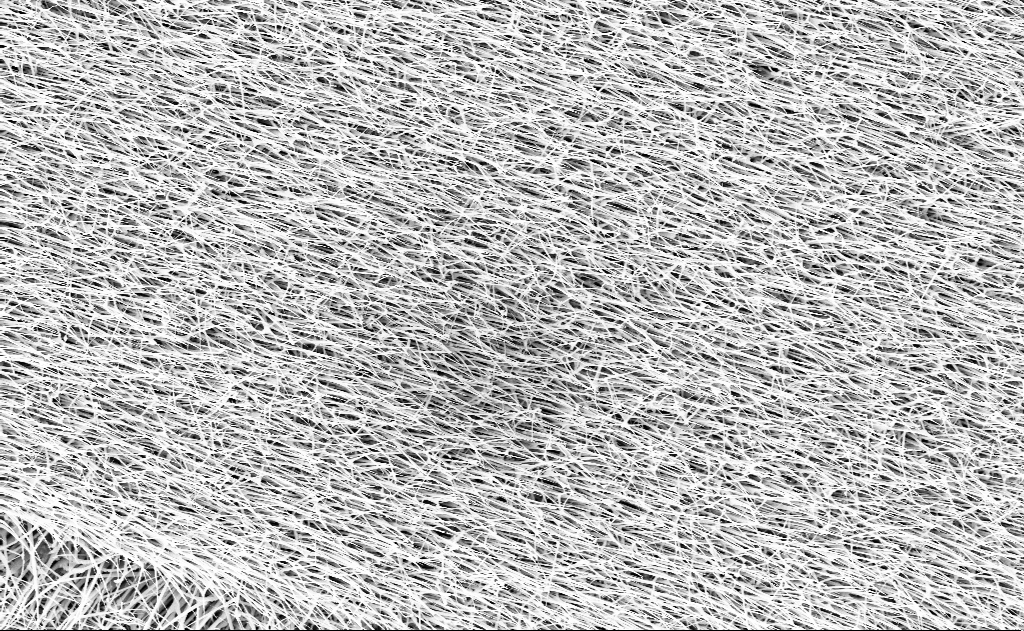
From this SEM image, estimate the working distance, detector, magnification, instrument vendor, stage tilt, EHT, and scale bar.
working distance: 13 mm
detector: InLens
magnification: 10 K X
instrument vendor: Zeiss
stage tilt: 0°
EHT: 10 kV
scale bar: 2000 nm